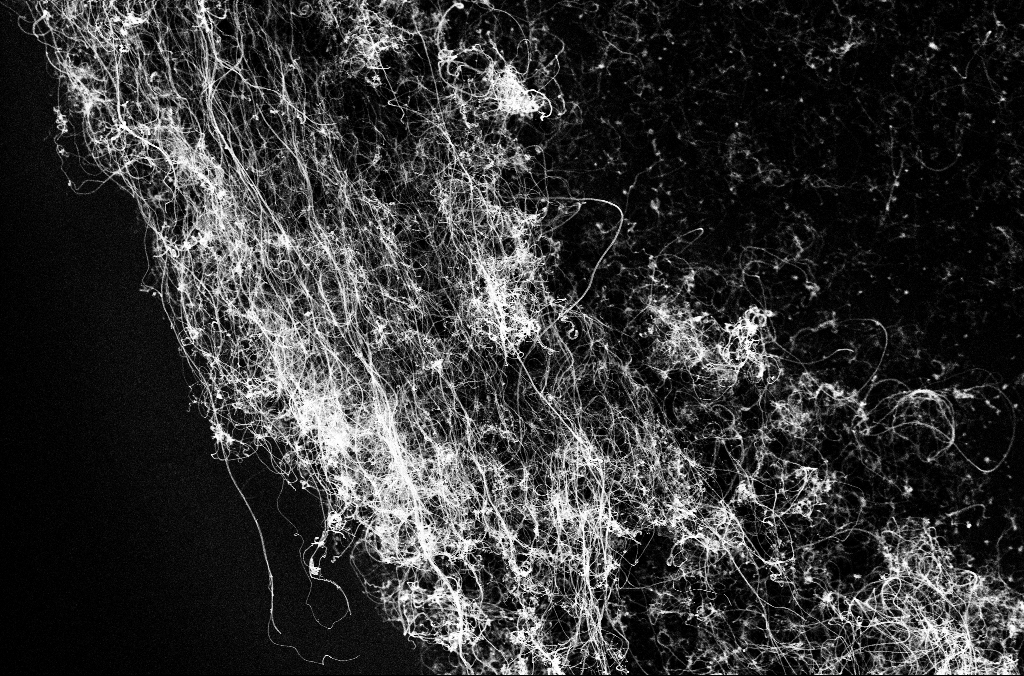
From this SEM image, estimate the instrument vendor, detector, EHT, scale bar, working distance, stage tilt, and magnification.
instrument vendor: Zeiss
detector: InLens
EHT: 10 kV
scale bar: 10000 nm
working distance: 3.3 mm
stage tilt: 0°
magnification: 6.31 K X